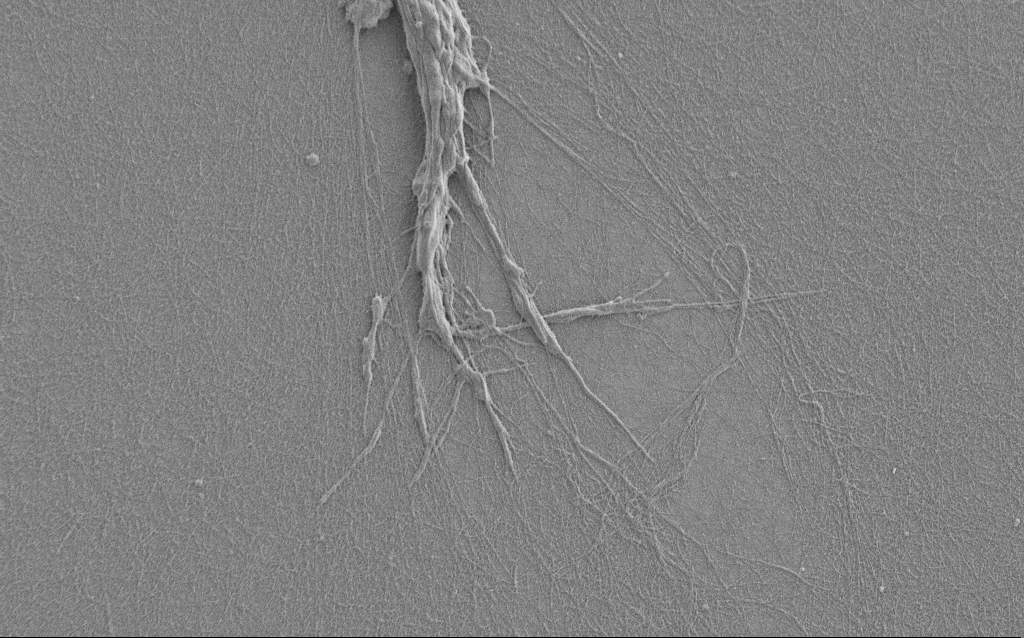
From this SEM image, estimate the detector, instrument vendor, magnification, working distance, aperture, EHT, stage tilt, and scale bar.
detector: SE2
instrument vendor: Zeiss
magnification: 7.5 K X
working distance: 6 mm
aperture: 30 µm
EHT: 1 kV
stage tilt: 0°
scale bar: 2000 nm